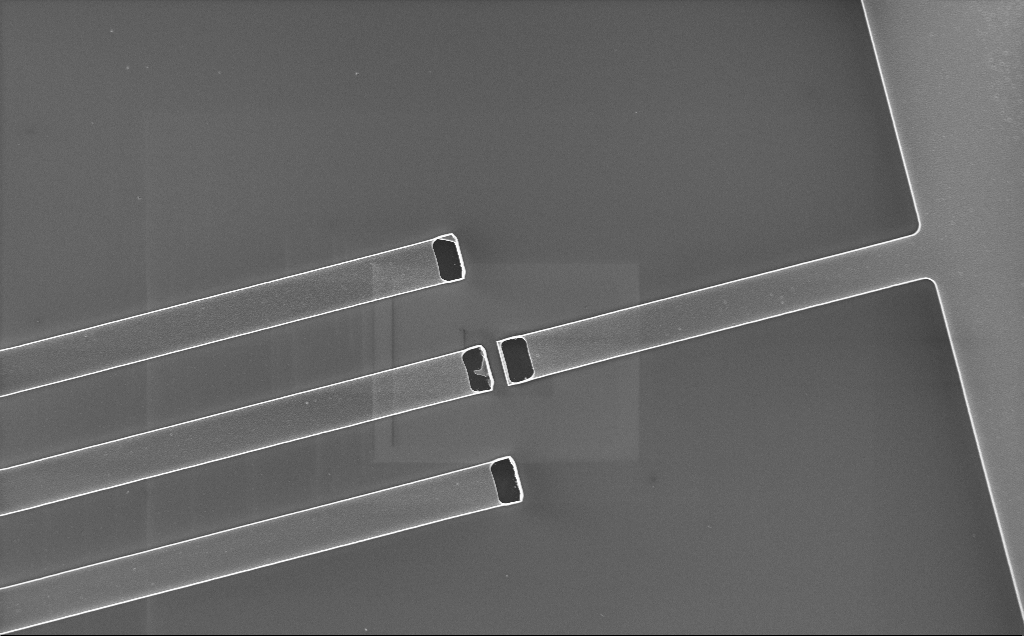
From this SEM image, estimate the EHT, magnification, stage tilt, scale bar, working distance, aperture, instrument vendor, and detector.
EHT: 2 kV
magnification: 1.06 K X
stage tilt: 0°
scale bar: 20000 nm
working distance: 10 mm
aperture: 30 µm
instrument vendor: Zeiss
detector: InLens